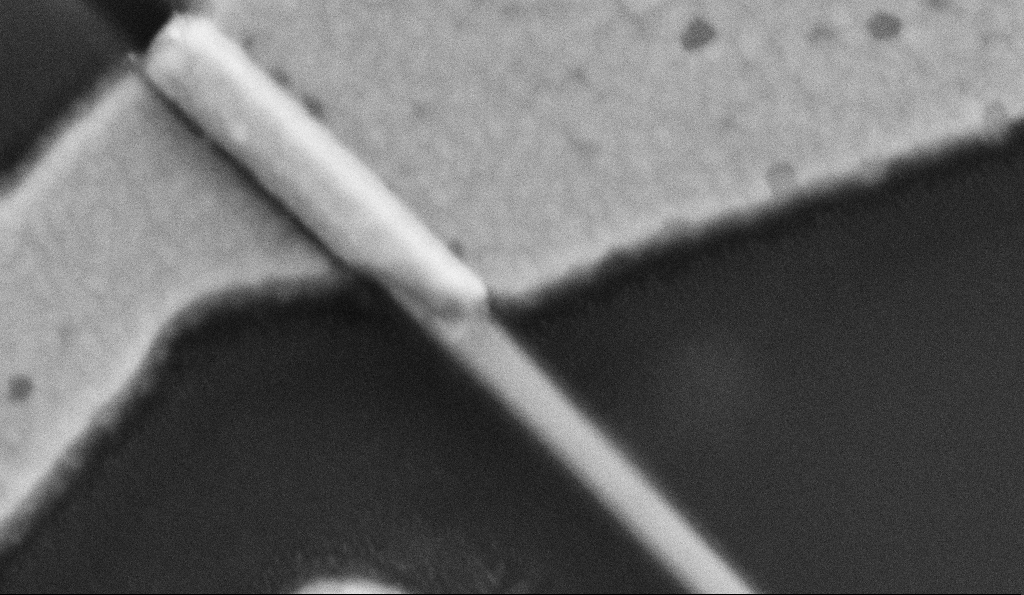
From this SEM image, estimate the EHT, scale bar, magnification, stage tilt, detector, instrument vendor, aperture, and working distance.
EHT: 5 kV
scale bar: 200 nm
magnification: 200 K X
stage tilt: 0°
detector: SE2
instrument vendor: Zeiss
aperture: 30 µm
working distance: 8.5 mm